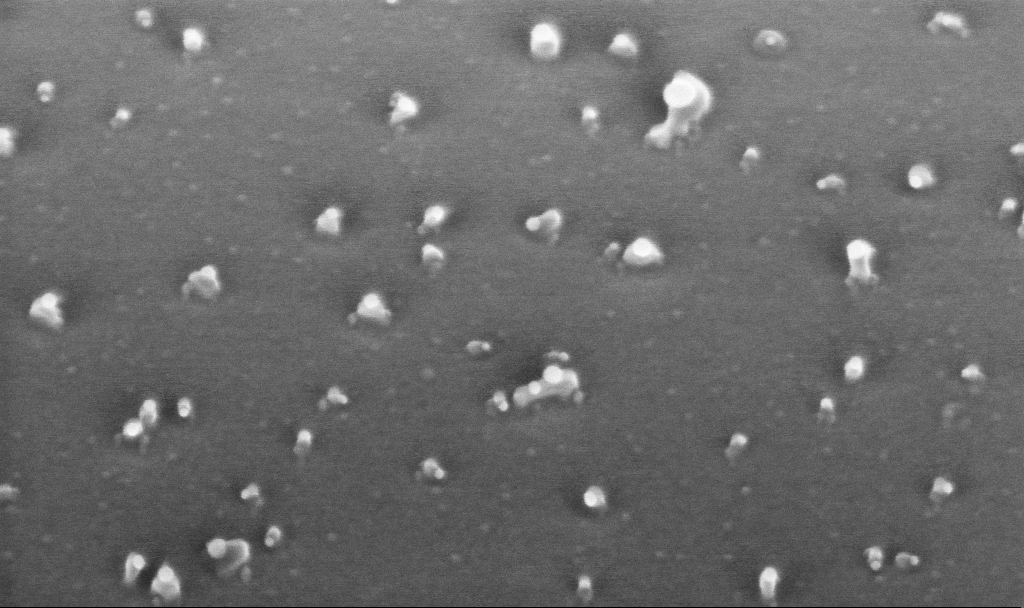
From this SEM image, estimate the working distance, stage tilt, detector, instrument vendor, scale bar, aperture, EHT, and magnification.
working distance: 4.4 mm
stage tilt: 45°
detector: InLens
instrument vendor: Zeiss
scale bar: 200 nm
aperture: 30 µm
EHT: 10 kV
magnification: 200 K X